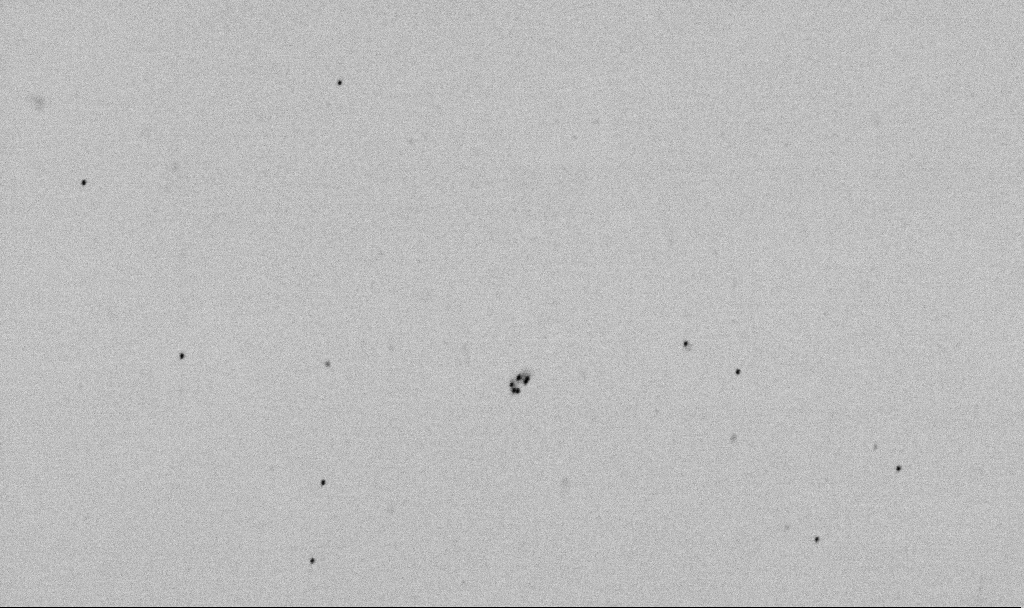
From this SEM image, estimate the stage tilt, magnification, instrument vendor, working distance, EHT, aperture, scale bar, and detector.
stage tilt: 0°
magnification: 50 K X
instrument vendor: Zeiss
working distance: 6.5 mm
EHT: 2 kV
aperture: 30 µm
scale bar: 1000 nm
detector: SE2